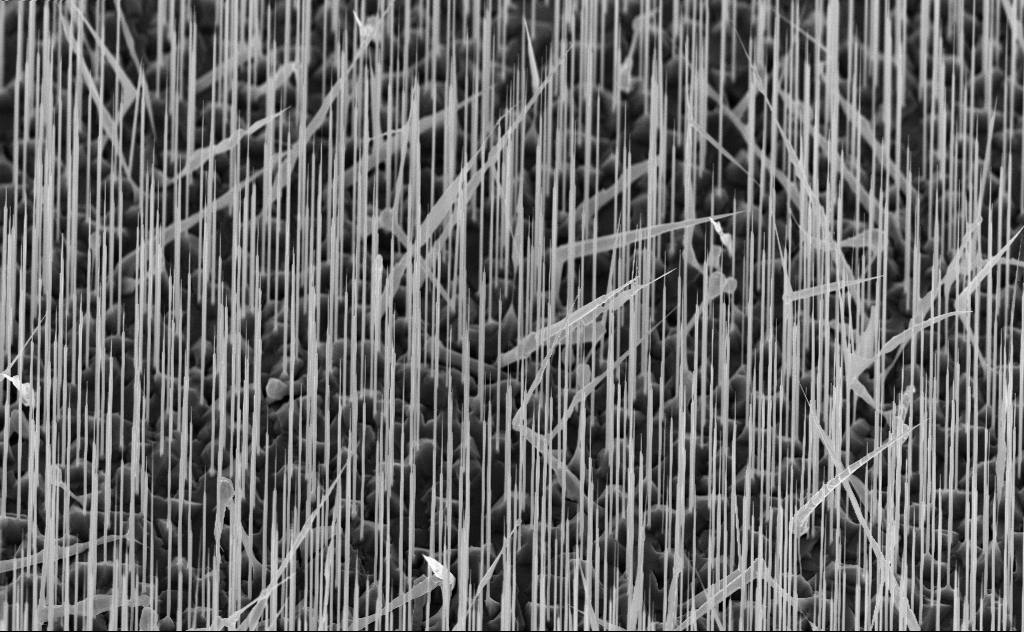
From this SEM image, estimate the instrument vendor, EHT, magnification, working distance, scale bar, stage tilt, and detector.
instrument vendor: Zeiss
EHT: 10 kV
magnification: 10 K X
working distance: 6 mm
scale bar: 2000 nm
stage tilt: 45°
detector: InLens